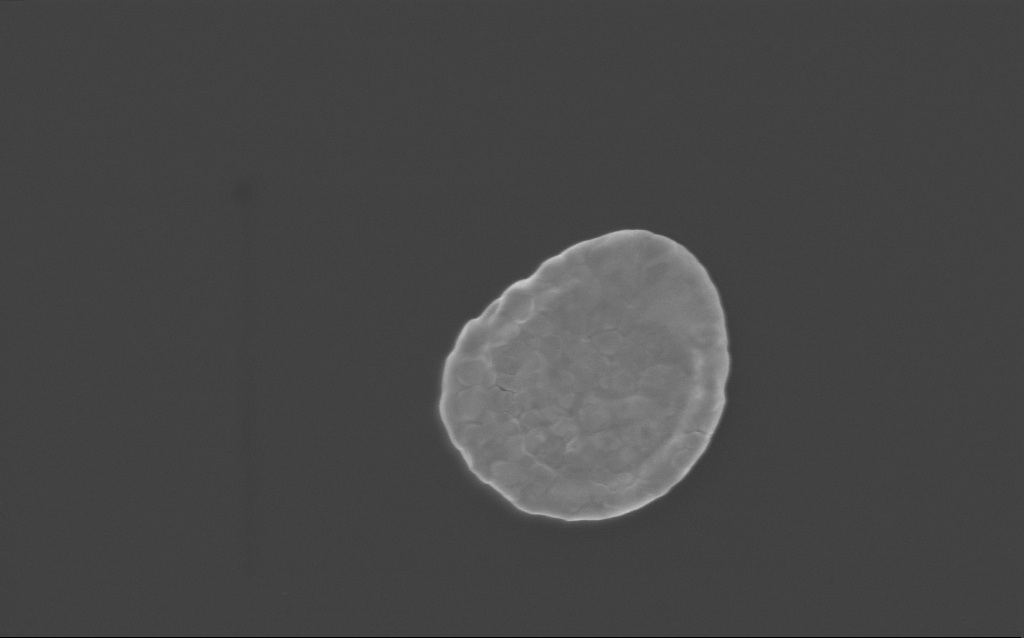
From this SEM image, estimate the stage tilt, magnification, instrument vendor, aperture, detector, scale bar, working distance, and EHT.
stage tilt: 0°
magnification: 45.13 K X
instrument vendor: Zeiss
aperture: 30 µm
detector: InLens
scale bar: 1000 nm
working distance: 3 mm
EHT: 2 kV